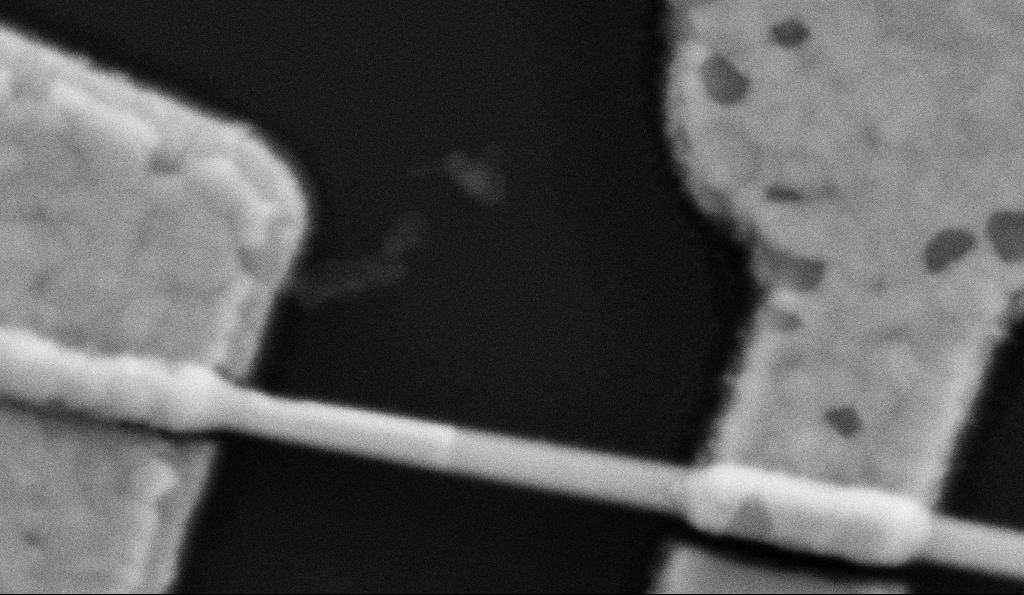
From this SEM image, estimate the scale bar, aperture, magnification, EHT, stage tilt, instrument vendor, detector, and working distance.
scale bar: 200 nm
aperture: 30 µm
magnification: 200 K X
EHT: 5 kV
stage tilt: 0°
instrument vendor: Zeiss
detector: SE2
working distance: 8.5 mm